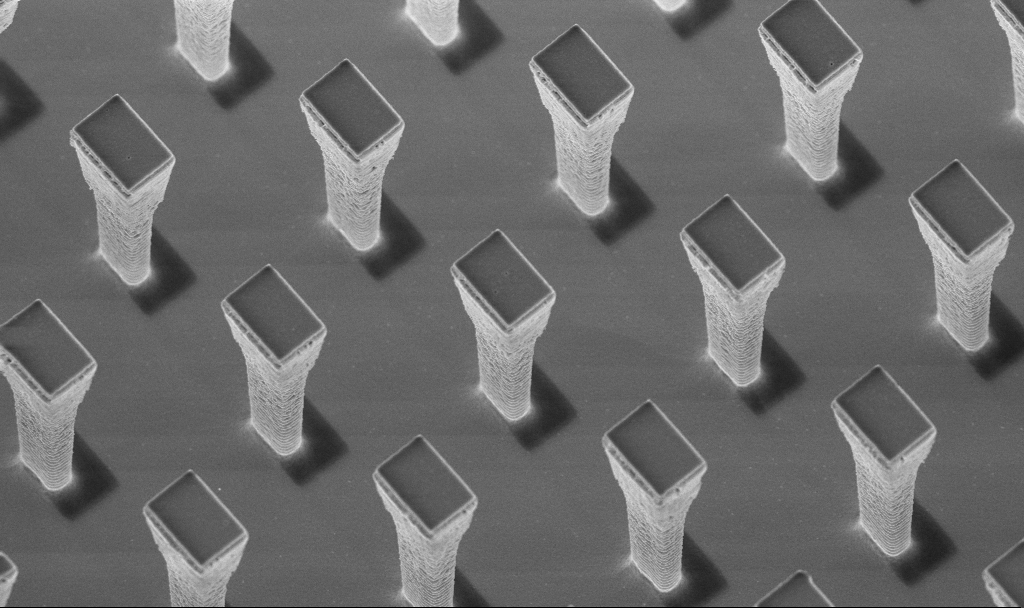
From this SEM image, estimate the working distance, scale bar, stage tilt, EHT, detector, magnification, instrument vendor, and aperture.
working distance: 4.3 mm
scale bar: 2000 nm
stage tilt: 20°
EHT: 5 kV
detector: InLens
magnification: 7.28 K X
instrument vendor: Zeiss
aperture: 30 µm